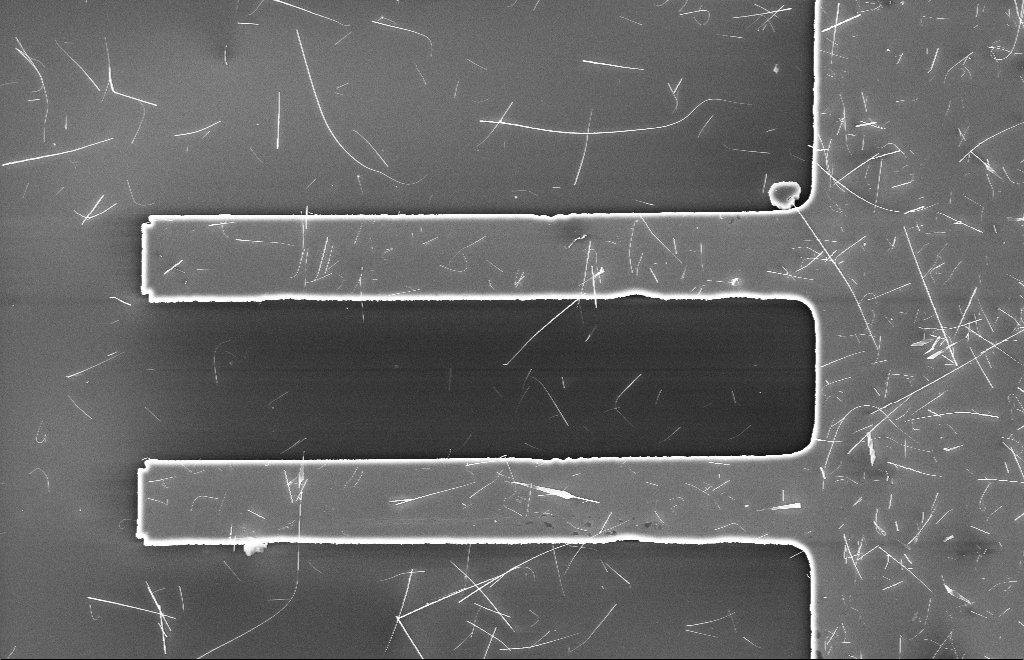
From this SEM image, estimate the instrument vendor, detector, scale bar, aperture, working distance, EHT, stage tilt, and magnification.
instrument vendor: Zeiss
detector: InLens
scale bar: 10000 nm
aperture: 20 µm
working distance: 6 mm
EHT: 10 kV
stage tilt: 0°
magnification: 2.5 K X